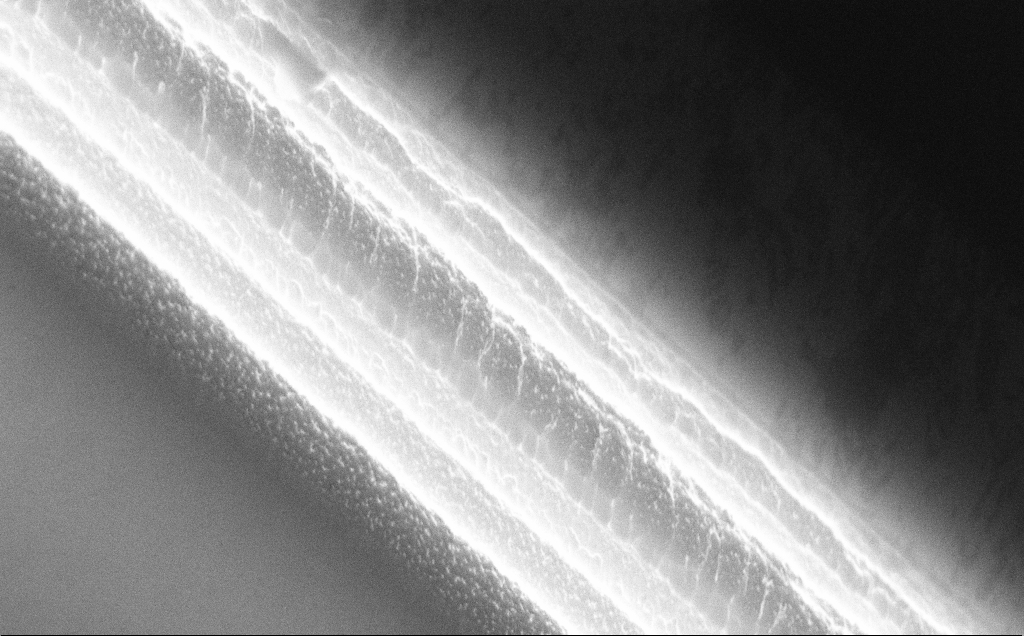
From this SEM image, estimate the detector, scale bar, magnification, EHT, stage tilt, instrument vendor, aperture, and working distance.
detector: InLens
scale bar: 1000 nm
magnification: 54.23 K X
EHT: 10 kV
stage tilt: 50°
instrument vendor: Zeiss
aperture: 30 µm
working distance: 9 mm